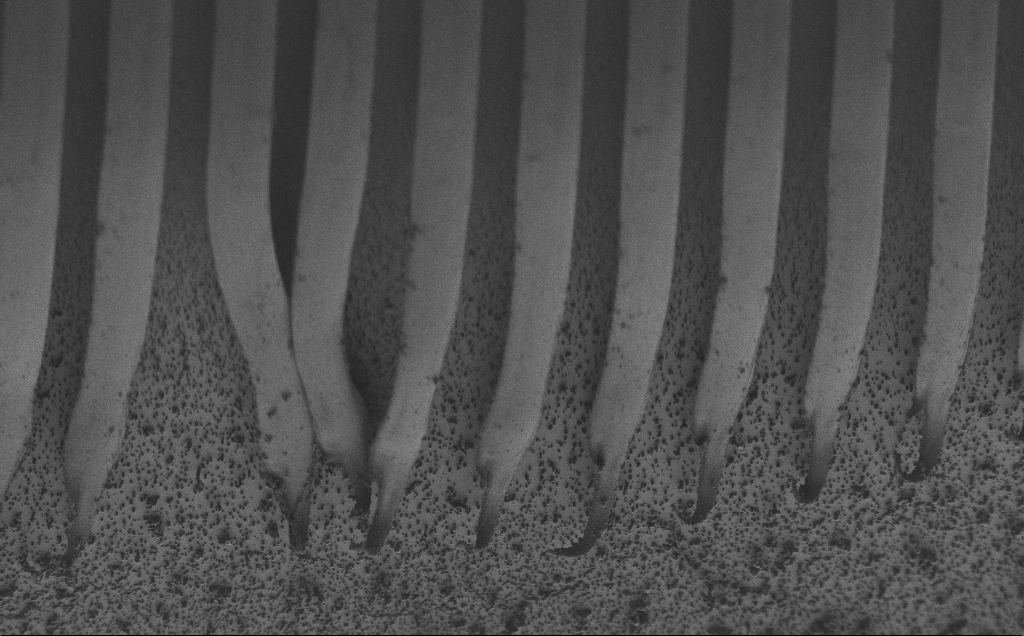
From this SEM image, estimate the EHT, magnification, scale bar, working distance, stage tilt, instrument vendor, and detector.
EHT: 1.2 kV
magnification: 1.19 K X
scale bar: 20000 nm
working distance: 5 mm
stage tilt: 45°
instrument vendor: Zeiss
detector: SE2